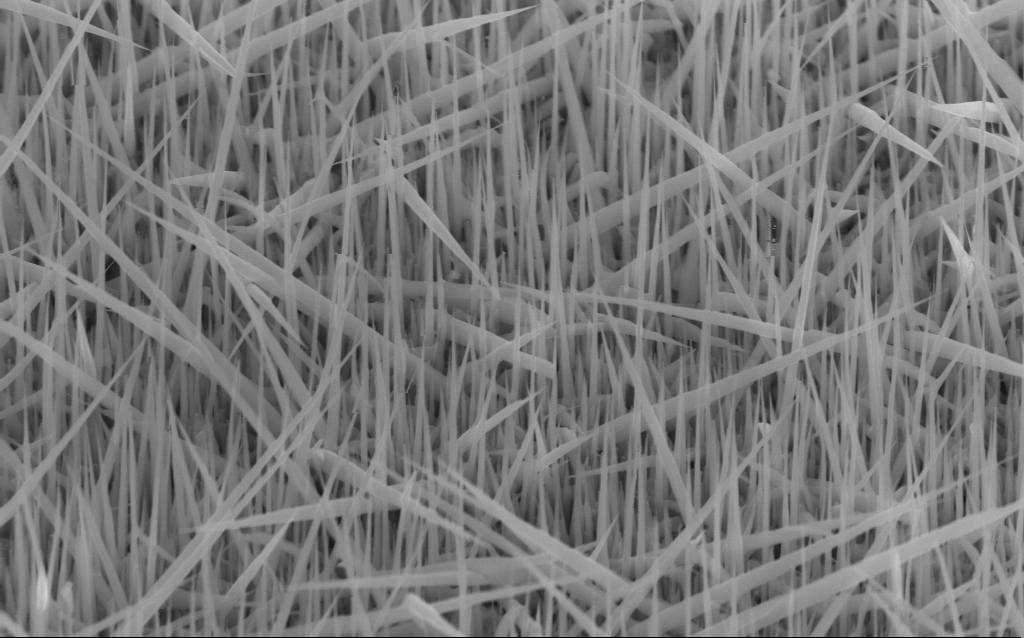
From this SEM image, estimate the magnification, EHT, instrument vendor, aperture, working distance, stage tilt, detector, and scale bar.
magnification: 45.29 K X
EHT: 10 kV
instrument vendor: Zeiss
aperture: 30 µm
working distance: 5 mm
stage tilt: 45.1°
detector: InLens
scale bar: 1000 nm